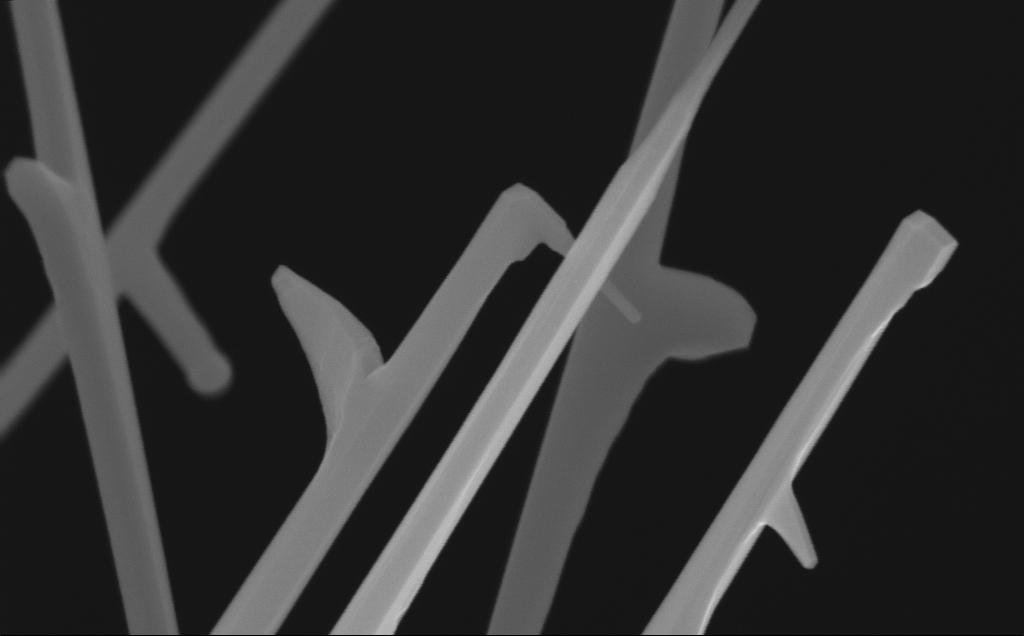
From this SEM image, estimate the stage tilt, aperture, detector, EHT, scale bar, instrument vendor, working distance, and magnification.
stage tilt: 0°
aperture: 30 µm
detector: InLens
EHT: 10 kV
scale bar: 200 nm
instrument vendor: Zeiss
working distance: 7 mm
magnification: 119.93 K X